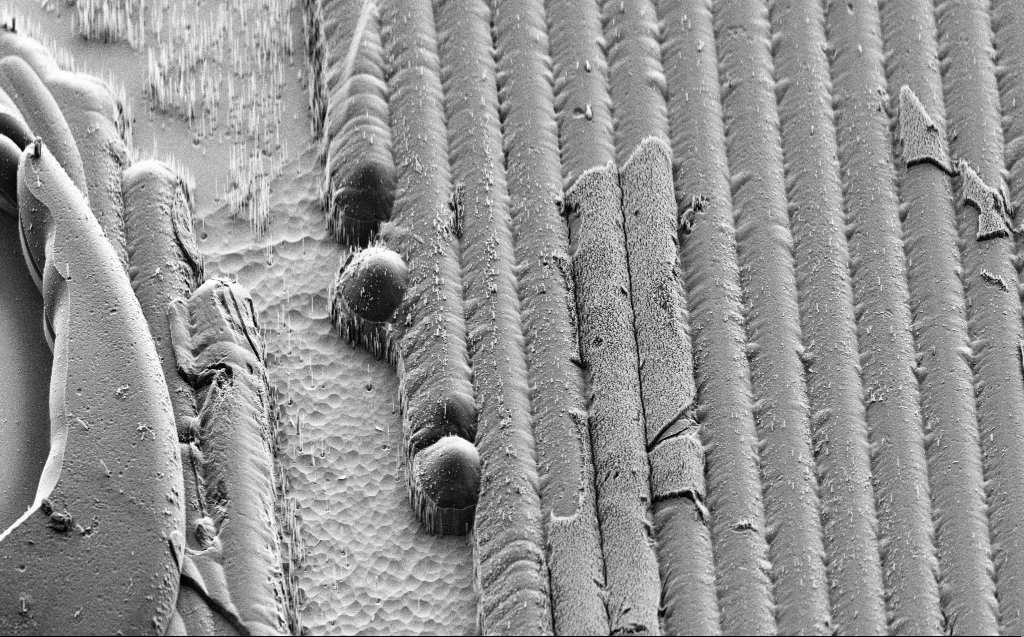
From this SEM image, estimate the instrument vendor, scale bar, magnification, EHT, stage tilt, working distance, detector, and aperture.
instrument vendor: Zeiss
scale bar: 20000 nm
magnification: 2.03 K X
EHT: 3 kV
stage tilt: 45°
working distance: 9 mm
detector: SE2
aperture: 30 µm